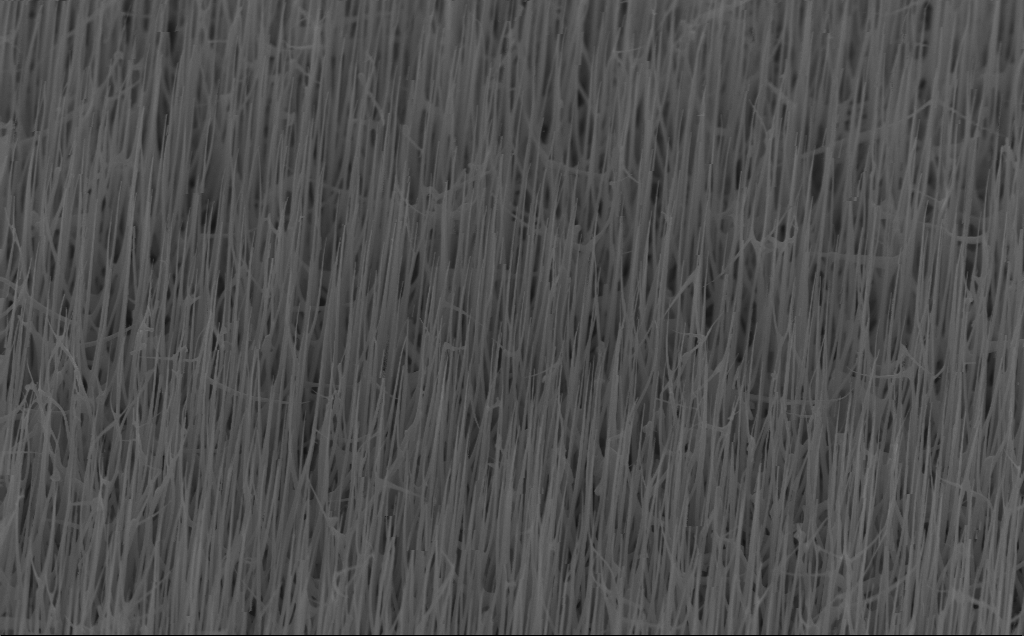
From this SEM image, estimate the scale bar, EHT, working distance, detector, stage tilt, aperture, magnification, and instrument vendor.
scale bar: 1000 nm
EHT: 10 kV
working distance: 6 mm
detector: InLens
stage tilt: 45°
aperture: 30 µm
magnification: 20 K X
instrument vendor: Zeiss